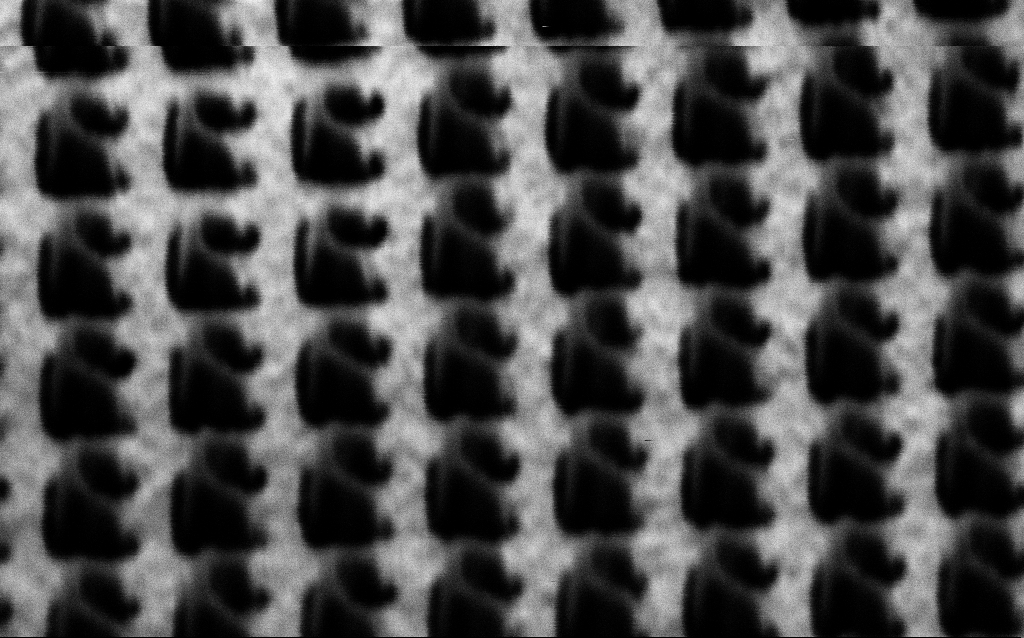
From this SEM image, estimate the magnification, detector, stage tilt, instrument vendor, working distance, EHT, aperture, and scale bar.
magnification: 100.72 K X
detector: SE2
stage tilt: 45°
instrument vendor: Zeiss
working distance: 7.8 mm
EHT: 1.5 kV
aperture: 30 µm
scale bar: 200 nm